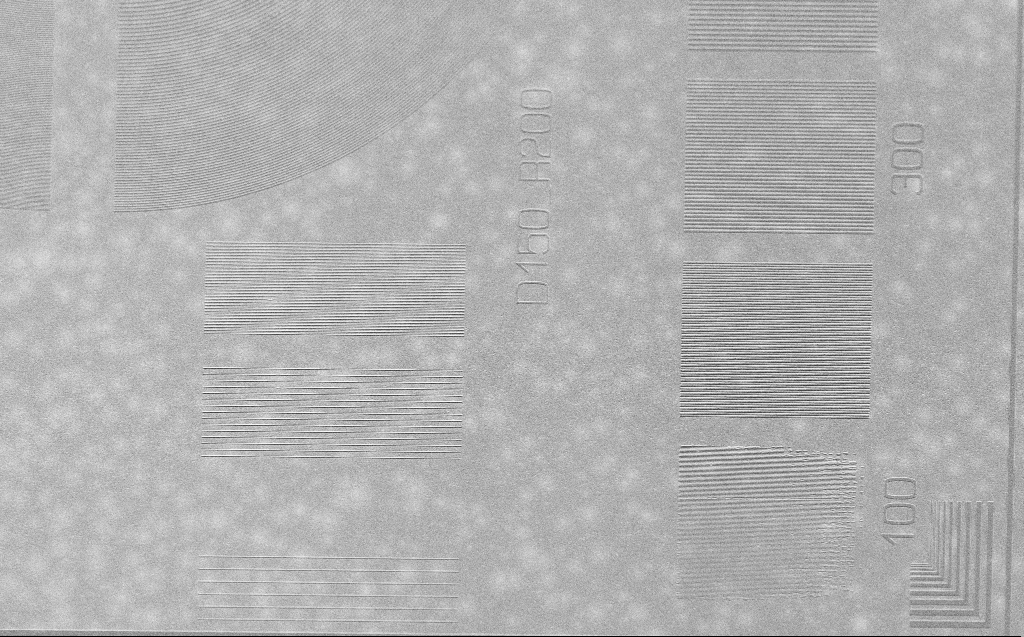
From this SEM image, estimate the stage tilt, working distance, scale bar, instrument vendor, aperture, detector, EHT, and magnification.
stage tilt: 30°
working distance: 4 mm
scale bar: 10000 nm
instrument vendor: Zeiss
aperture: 30 µm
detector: SE2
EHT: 2.5 kV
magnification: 2.36 K X